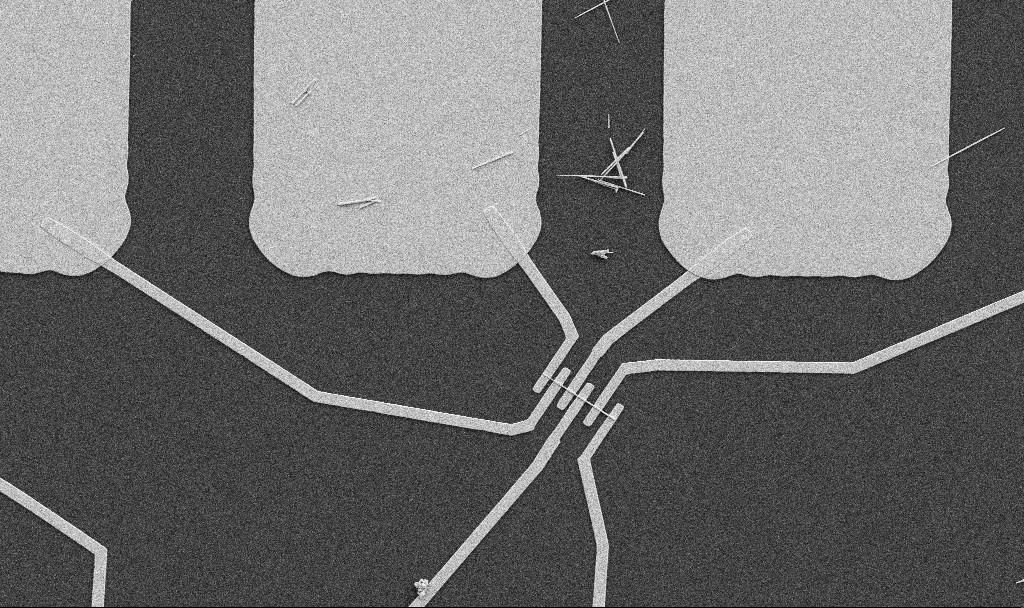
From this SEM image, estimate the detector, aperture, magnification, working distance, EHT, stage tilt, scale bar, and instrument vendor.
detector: SE2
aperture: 30 µm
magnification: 5 K X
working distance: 10.7 mm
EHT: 5 kV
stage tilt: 0°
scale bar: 10000 nm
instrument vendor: Zeiss